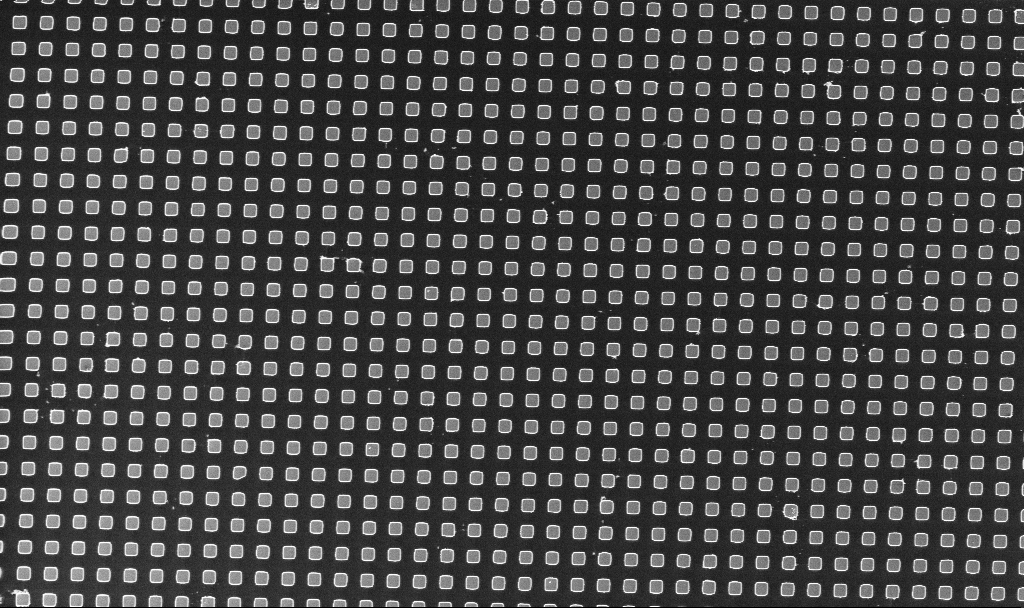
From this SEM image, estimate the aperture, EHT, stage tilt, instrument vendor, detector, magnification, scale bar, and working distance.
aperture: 30 µm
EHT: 3 kV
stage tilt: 0°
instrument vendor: Zeiss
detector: InLens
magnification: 2.44 K X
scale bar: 10000 nm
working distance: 2.8 mm